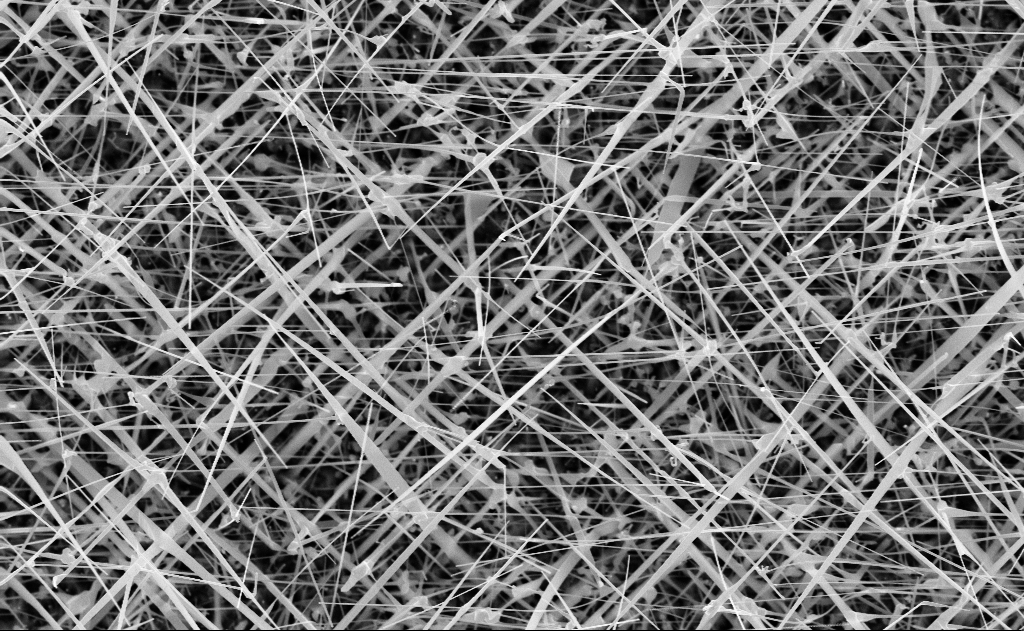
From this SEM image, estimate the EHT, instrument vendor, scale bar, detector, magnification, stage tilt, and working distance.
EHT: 10 kV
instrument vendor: Zeiss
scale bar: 1000 nm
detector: InLens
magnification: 20 K X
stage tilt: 0°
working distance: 11 mm